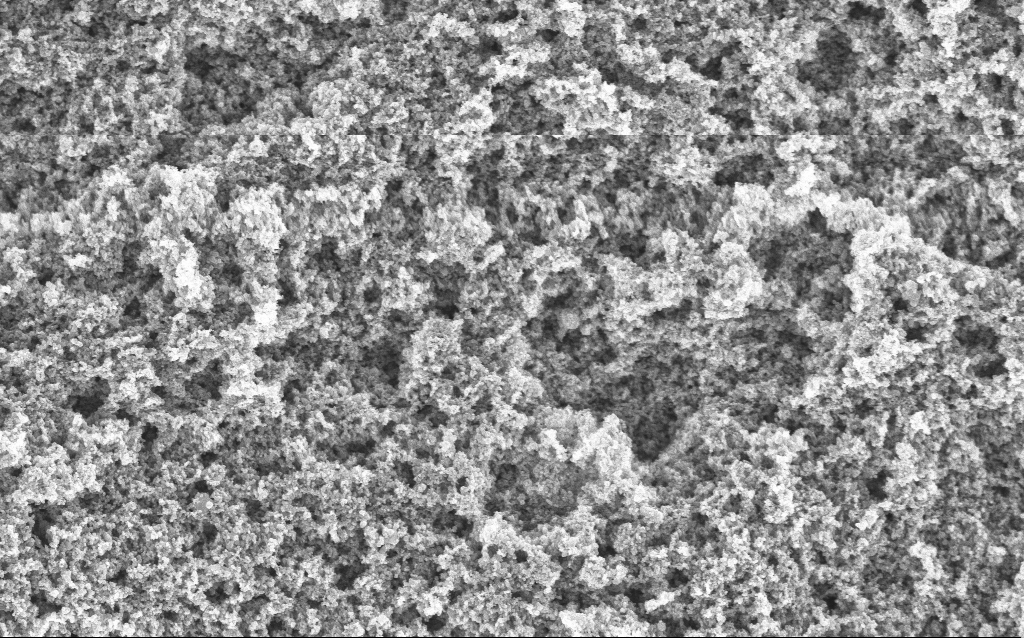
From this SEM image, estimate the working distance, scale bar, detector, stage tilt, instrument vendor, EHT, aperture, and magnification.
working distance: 4.4 mm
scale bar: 1000 nm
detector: InLens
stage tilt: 0°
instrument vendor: Zeiss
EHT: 5 kV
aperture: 30 µm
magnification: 37.88 K X